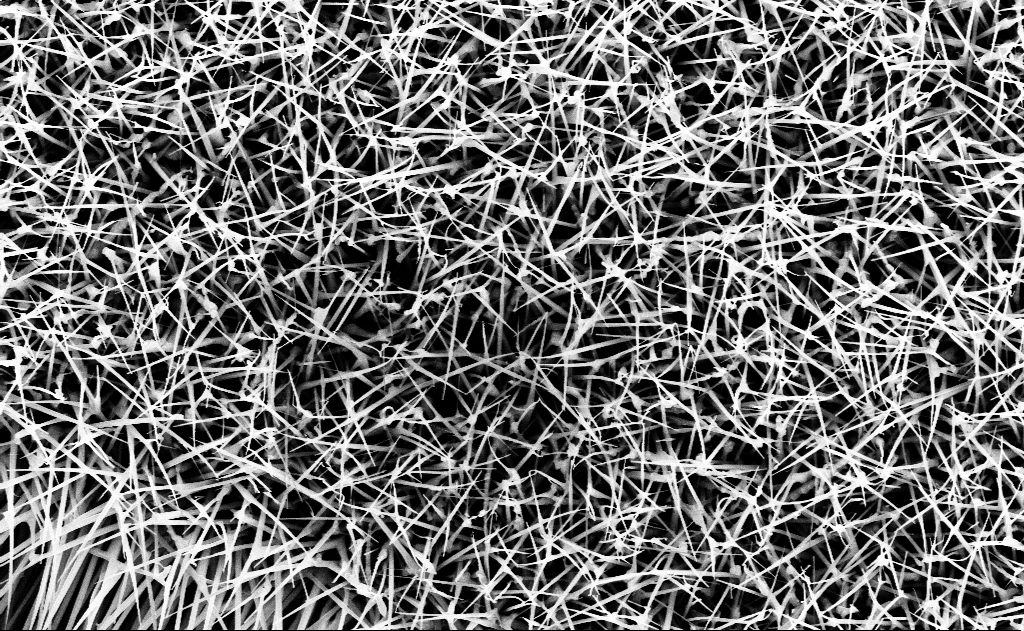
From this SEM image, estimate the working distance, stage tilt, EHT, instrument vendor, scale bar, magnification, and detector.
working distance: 13 mm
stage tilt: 0°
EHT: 10 kV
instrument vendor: Zeiss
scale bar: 2000 nm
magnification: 20 K X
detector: InLens